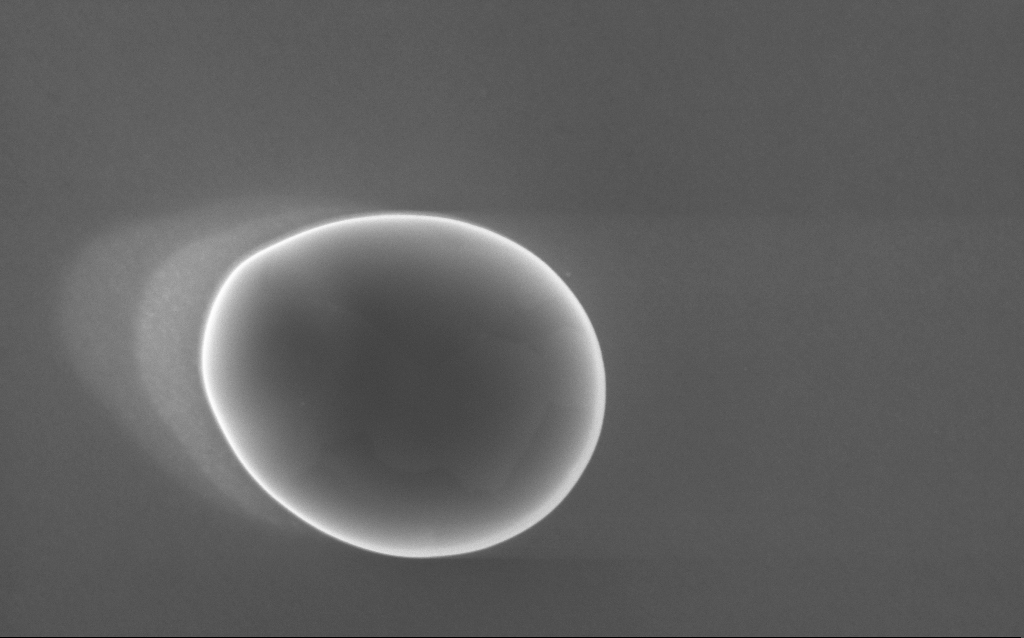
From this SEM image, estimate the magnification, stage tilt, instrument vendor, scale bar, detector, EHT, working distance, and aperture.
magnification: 90.36 K X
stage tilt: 0°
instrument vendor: Zeiss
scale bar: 200 nm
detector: InLens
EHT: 10 kV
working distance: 3 mm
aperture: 30 µm